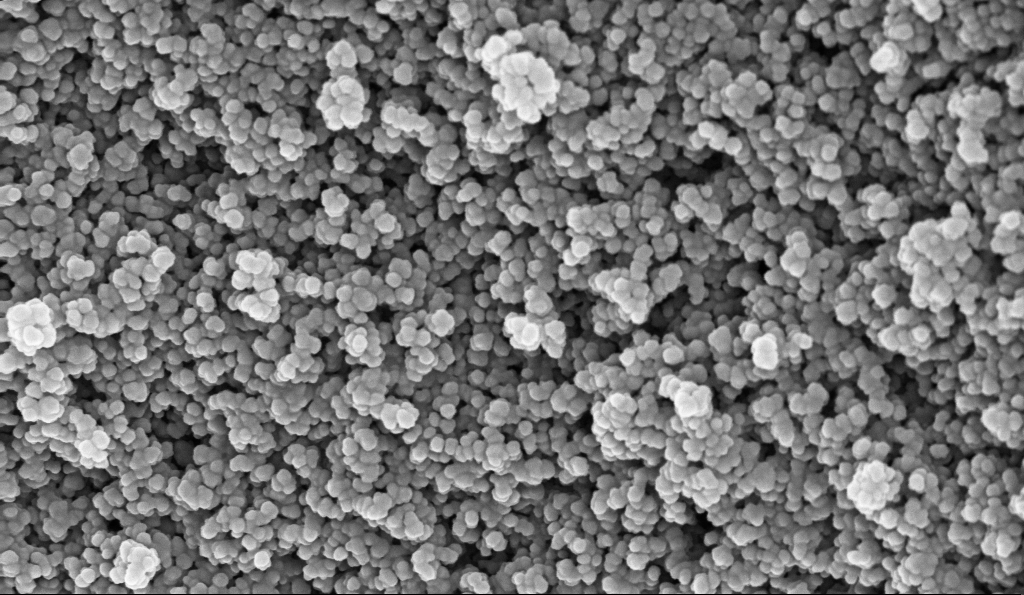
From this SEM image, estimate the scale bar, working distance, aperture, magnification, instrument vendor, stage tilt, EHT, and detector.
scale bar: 100 nm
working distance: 5.1 mm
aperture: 30 µm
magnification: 135 K X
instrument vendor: Zeiss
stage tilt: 0°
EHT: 10 kV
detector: InLens